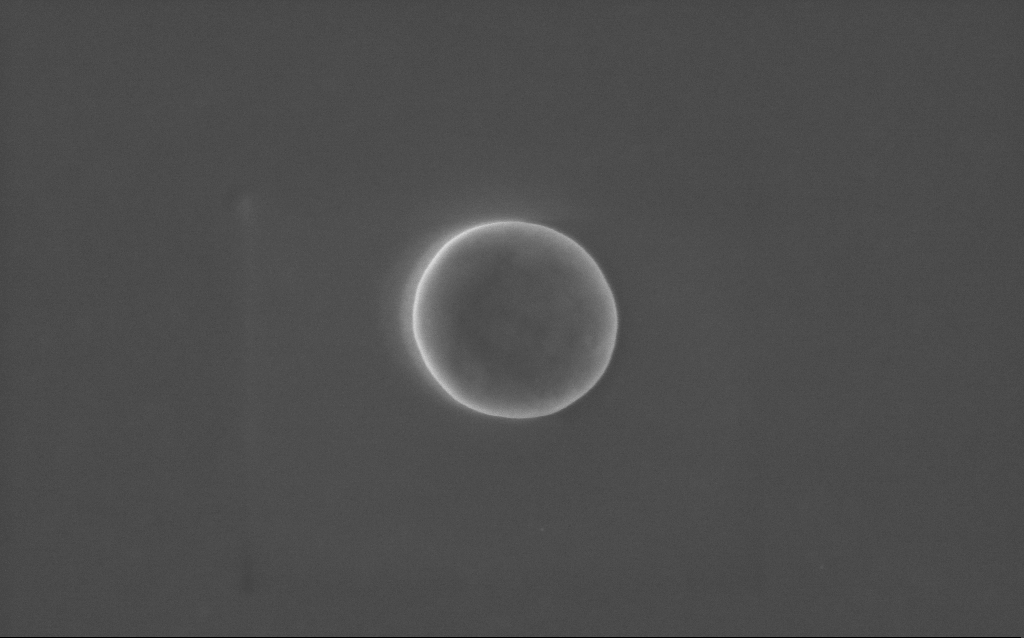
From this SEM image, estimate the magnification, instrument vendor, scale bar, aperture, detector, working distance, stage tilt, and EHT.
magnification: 62.21 K X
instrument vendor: Zeiss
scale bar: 1000 nm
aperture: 30 µm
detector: InLens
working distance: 2 mm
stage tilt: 0°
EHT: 5 kV